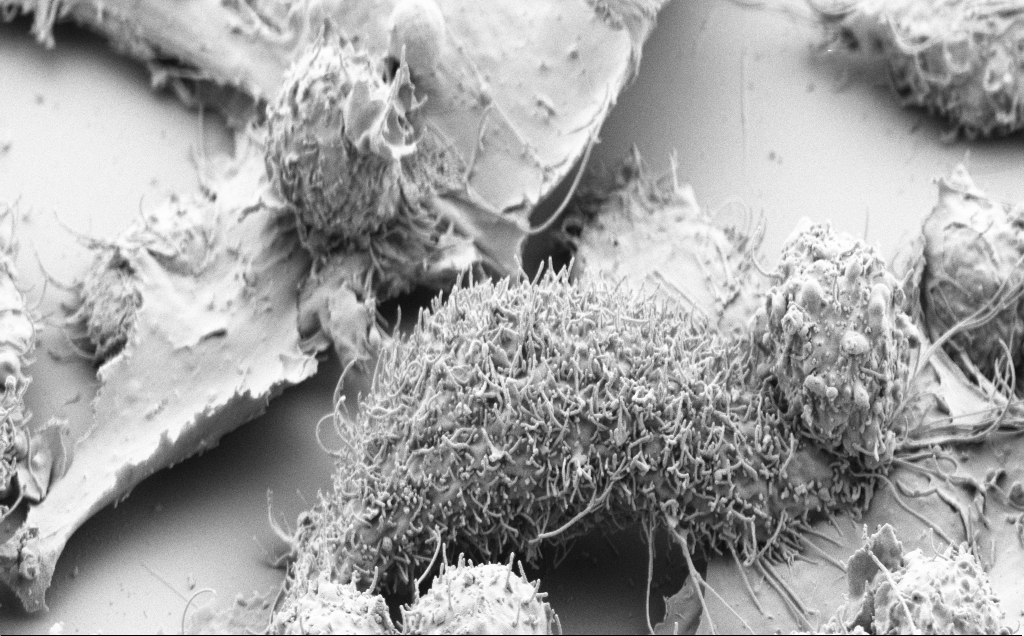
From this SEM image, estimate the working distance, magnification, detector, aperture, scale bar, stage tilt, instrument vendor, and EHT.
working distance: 7 mm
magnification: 6.79 K X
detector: SE2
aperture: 30 µm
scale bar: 10000 nm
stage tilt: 46.1°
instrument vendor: Zeiss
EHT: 3 kV